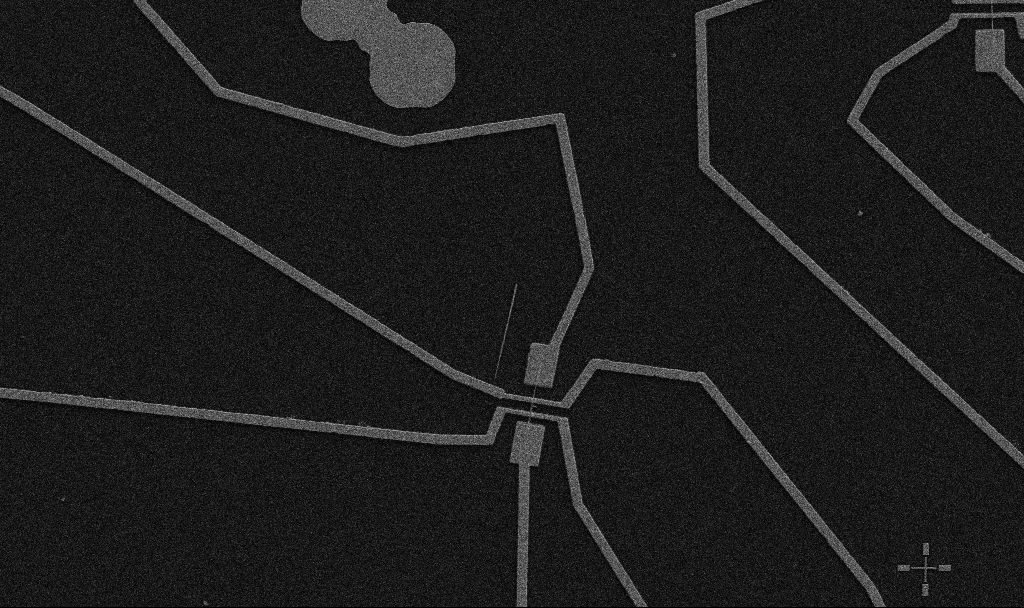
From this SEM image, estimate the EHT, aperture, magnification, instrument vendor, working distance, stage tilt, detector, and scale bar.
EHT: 5 kV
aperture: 30 µm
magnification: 5 K X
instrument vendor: Zeiss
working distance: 10.7 mm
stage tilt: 0°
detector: SE2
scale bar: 10000 nm